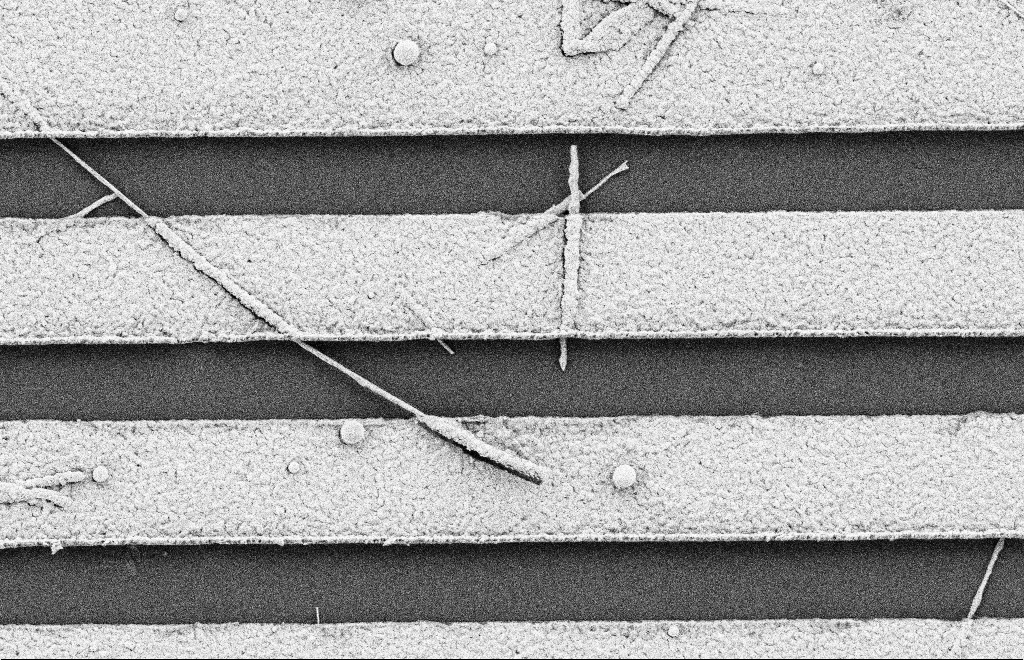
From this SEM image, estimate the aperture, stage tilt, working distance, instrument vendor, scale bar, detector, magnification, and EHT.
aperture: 20 µm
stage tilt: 0°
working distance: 10 mm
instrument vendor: Zeiss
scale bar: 2000 nm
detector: SE2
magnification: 18.73 K X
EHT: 2 kV